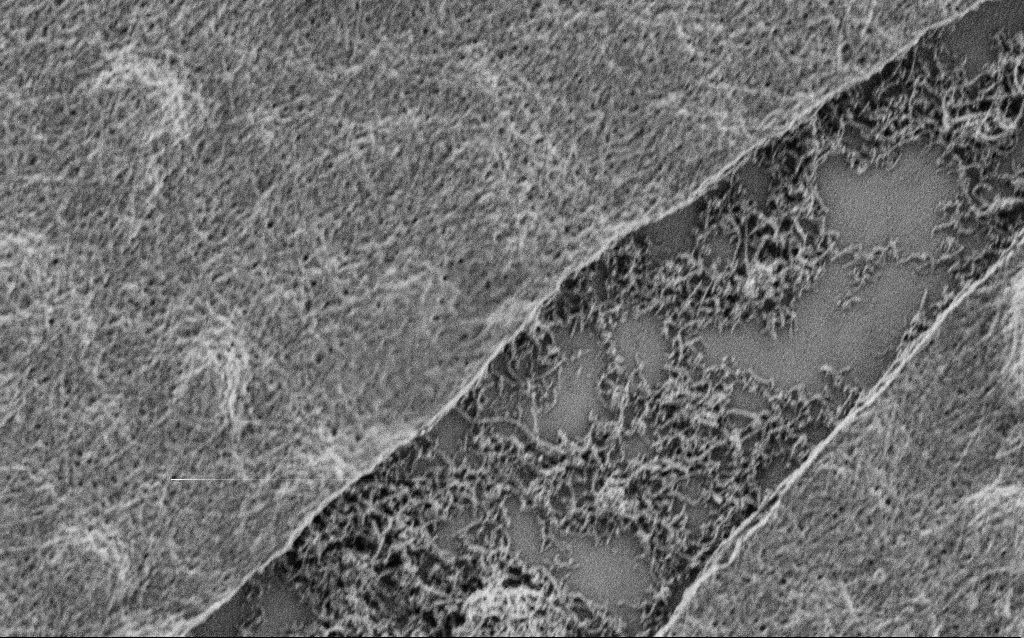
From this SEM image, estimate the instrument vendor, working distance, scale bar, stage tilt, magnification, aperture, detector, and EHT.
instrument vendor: Zeiss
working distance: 4 mm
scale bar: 1000 nm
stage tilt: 0°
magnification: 20 K X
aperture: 30 µm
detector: SE2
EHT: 1 kV